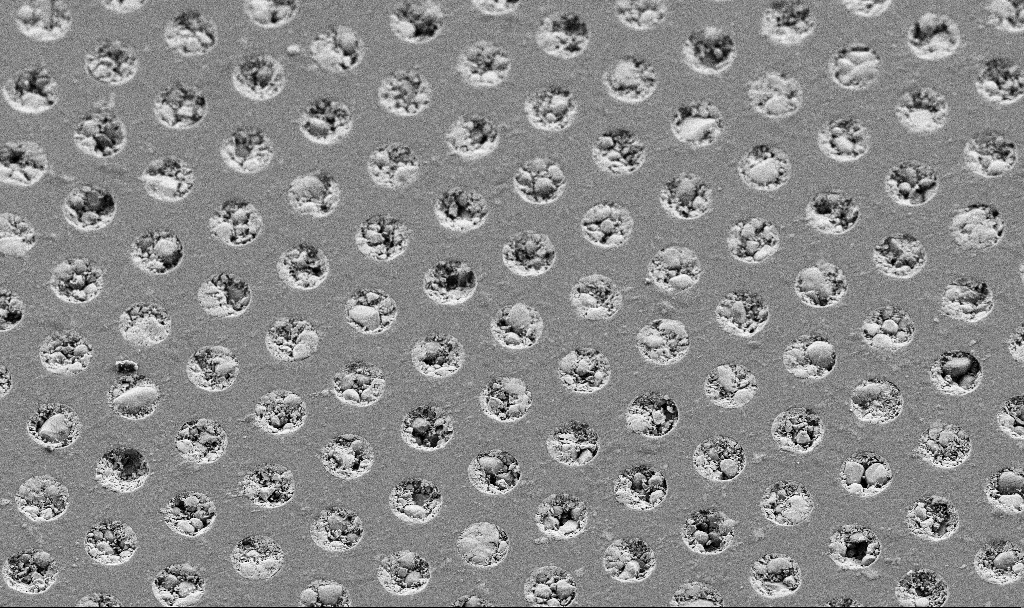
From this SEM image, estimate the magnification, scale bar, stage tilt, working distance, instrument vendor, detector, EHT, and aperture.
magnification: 5.23 K X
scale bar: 10000 nm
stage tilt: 30°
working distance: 4.5 mm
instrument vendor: Zeiss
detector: SE2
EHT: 3 kV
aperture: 30 µm